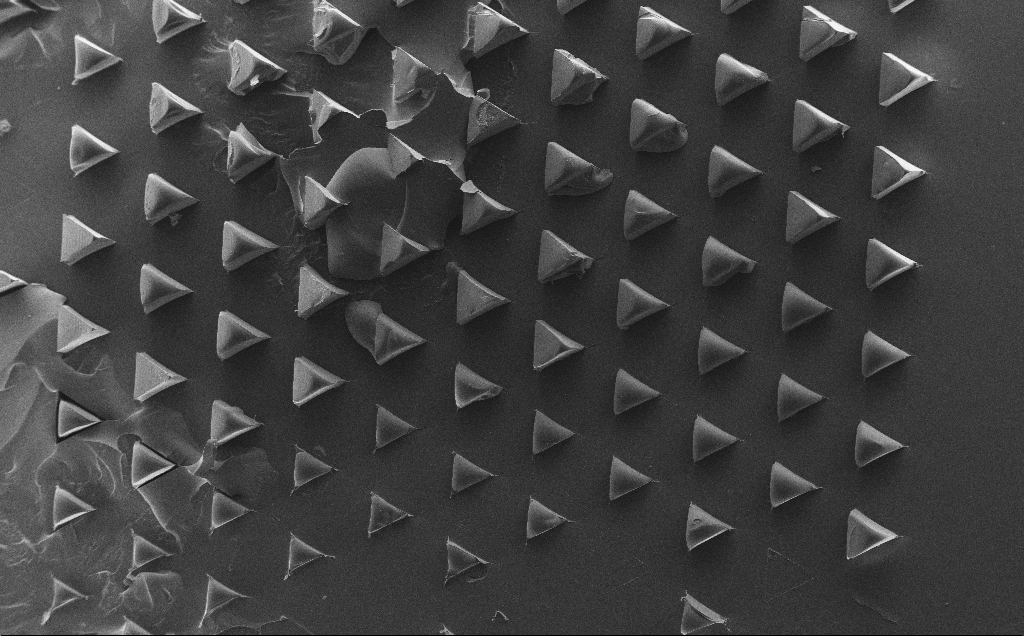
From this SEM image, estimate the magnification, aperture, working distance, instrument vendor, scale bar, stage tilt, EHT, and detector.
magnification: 0.074 K X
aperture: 30 µm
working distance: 11 mm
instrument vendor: Zeiss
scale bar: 200000 nm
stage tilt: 0°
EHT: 10 kV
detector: SE2